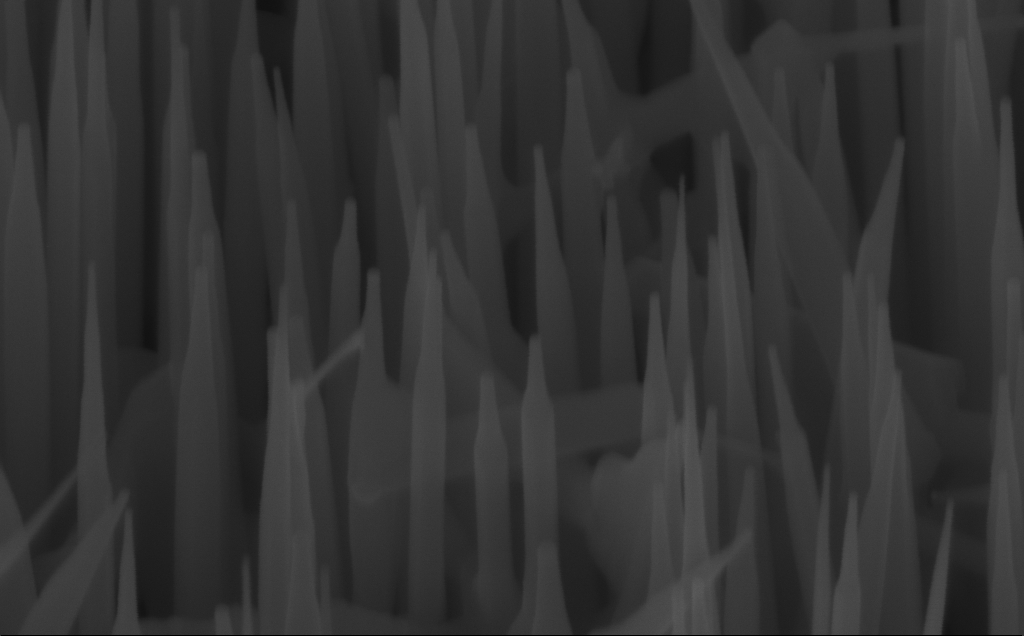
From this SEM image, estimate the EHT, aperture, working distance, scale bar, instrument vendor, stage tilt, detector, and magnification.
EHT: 10 kV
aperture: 30 µm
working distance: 7 mm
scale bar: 200 nm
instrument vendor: Zeiss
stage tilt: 45°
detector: InLens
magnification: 150 K X